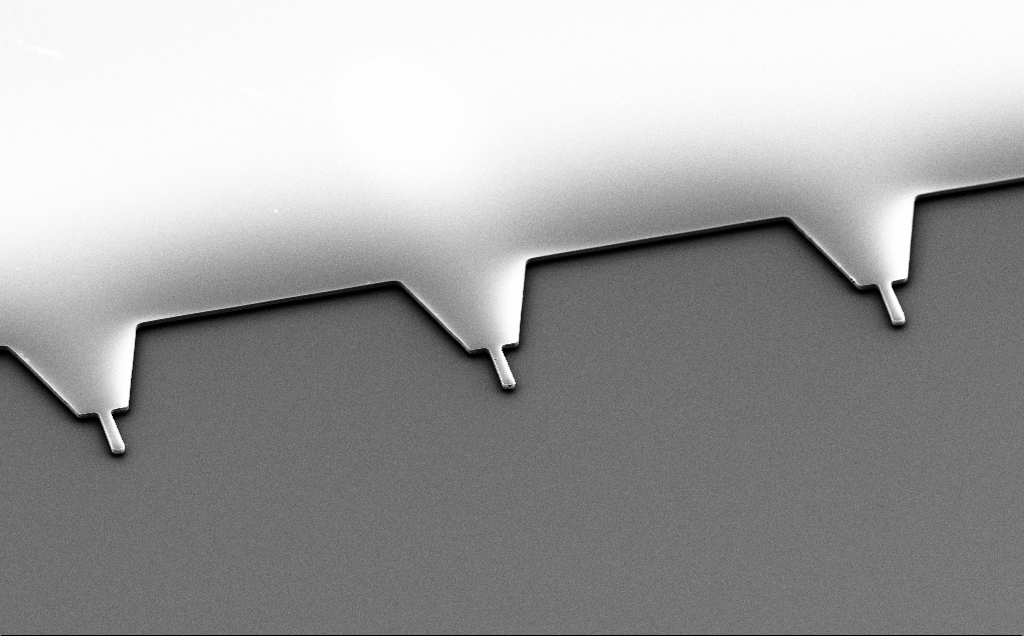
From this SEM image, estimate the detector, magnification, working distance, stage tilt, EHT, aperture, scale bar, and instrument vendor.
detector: SE2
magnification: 0.929 K X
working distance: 10 mm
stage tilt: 50°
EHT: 5 kV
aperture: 30 µm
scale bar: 20000 nm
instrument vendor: Zeiss